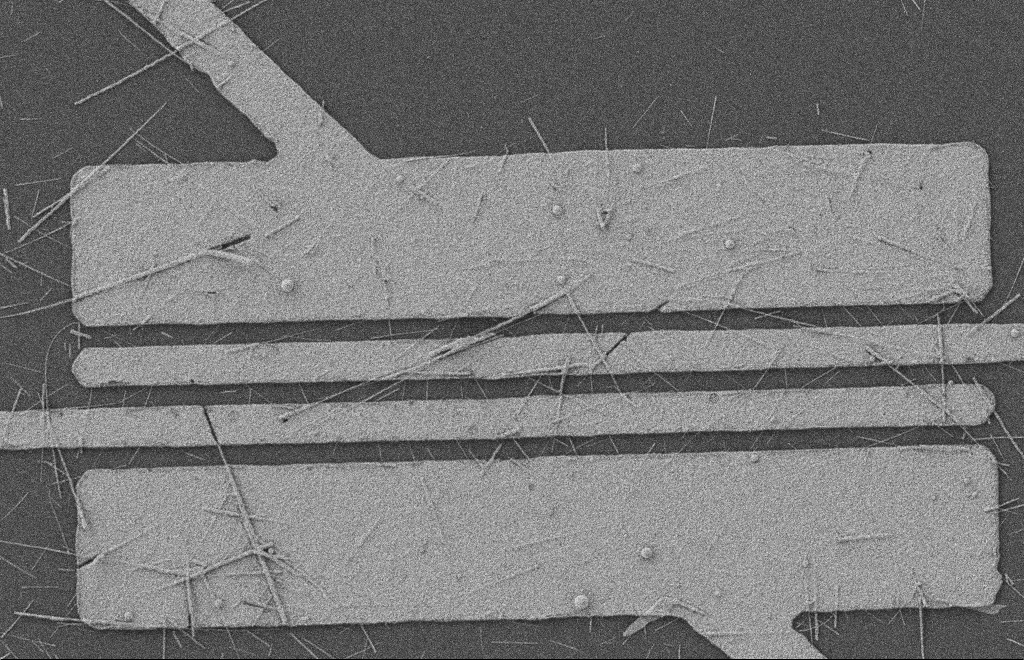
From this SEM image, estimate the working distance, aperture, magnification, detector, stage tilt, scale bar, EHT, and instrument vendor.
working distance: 8 mm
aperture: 20 µm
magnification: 5.56 K X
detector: SE2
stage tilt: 0°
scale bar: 2000 nm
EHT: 2 kV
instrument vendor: Zeiss